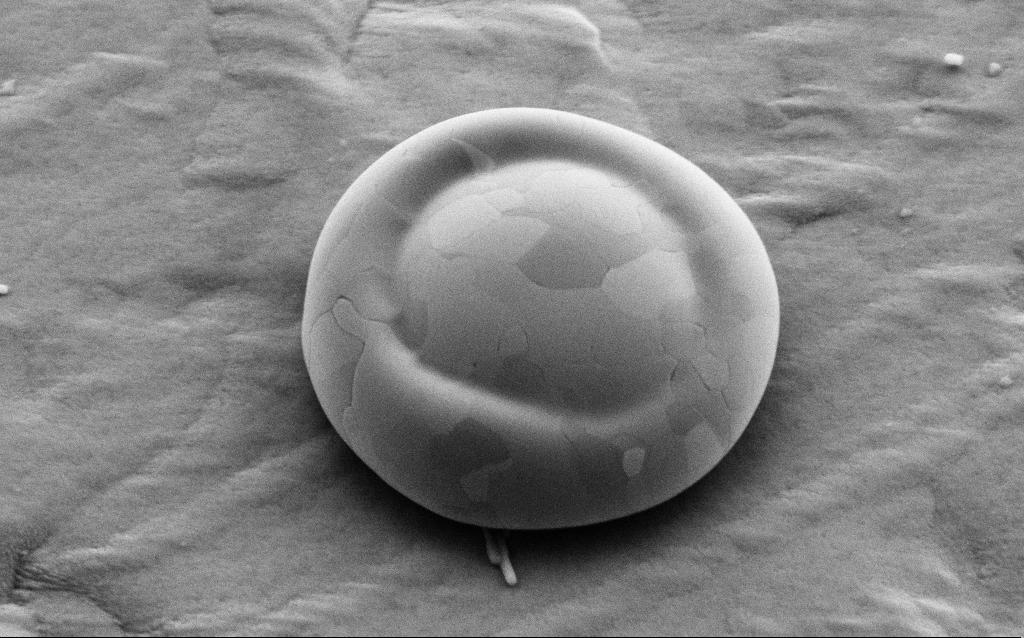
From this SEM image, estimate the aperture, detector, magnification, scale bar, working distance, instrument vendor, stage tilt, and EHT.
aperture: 30 µm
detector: SE2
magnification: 41.93 K X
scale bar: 1000 nm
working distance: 4 mm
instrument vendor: Zeiss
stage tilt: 35°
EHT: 5 kV